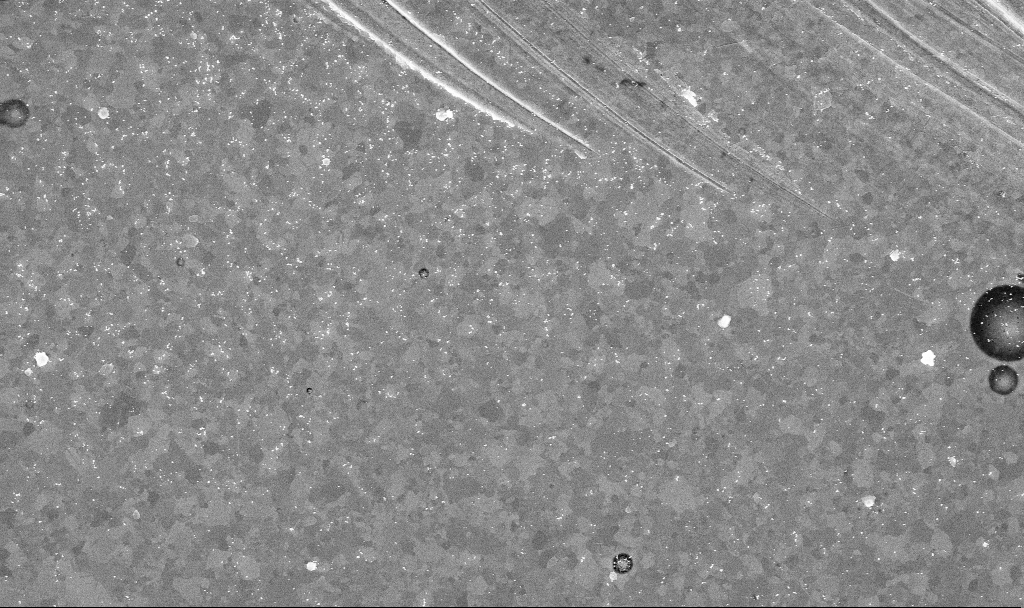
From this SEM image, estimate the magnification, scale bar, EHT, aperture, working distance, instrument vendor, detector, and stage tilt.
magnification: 20.42 K X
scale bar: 1000 nm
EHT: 10 kV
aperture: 30 µm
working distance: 3.4 mm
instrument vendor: Zeiss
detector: InLens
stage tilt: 0°